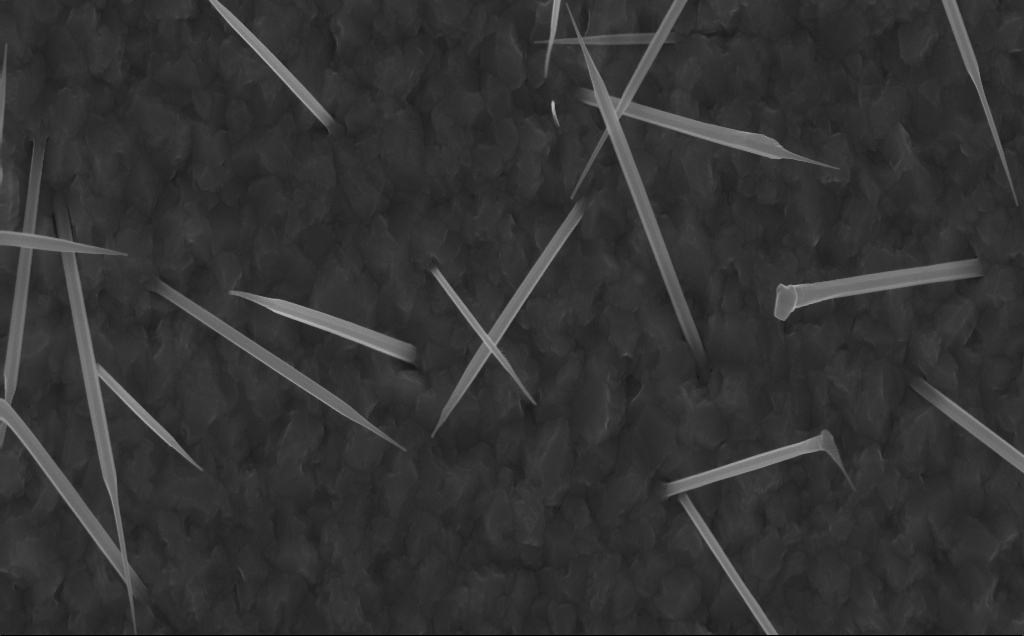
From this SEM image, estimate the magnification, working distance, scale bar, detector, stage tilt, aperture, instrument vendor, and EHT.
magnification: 40 K X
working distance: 5 mm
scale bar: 1000 nm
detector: InLens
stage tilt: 0°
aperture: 30 µm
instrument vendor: Zeiss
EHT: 10 kV